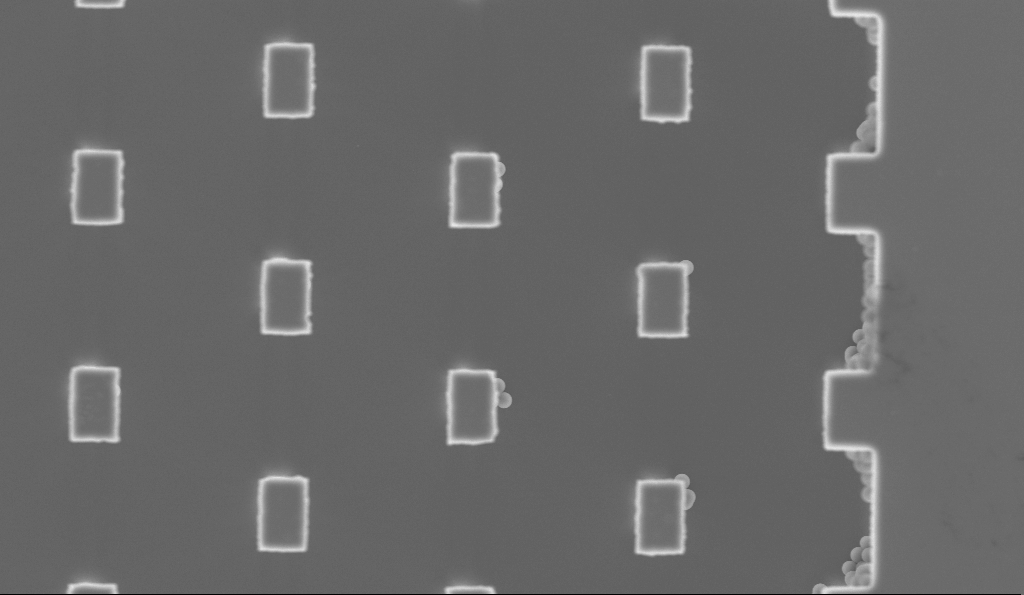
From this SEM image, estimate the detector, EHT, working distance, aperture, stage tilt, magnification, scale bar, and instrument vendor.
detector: InLens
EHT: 5 kV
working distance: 3.1 mm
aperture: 30 µm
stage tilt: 0°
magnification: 9.98 K X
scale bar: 2000 nm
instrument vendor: Zeiss